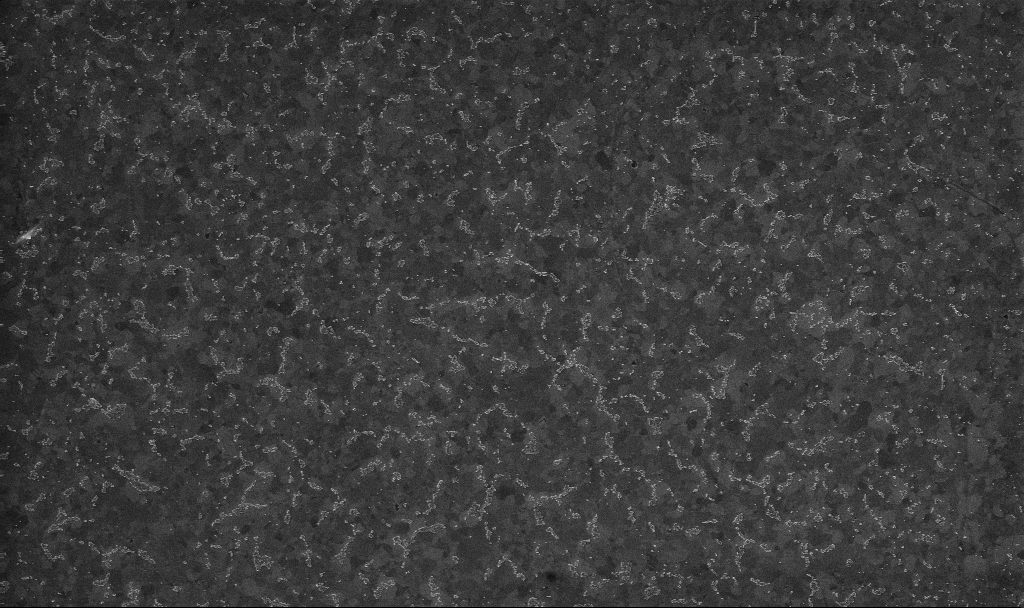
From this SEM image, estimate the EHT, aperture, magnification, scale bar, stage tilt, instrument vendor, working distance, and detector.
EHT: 10 kV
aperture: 30 µm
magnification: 20 K X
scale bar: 1000 nm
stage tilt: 0°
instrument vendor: Zeiss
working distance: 3.2 mm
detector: InLens